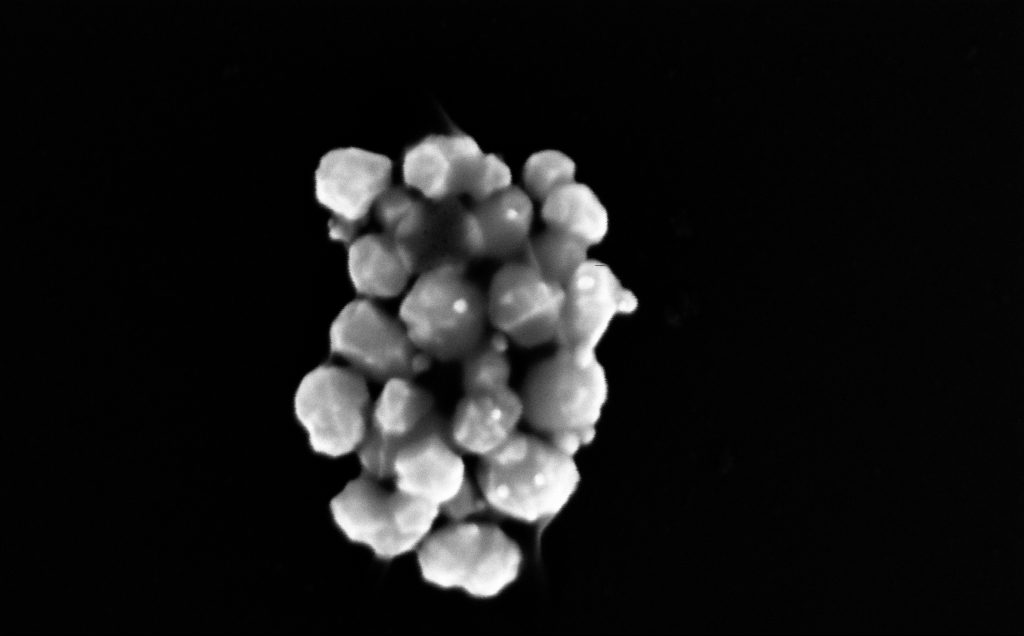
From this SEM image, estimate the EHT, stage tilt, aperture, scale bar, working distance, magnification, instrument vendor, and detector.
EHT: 10 kV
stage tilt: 0°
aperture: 30 µm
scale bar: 200 nm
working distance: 4 mm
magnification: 300.17 K X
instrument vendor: Zeiss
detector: InLens